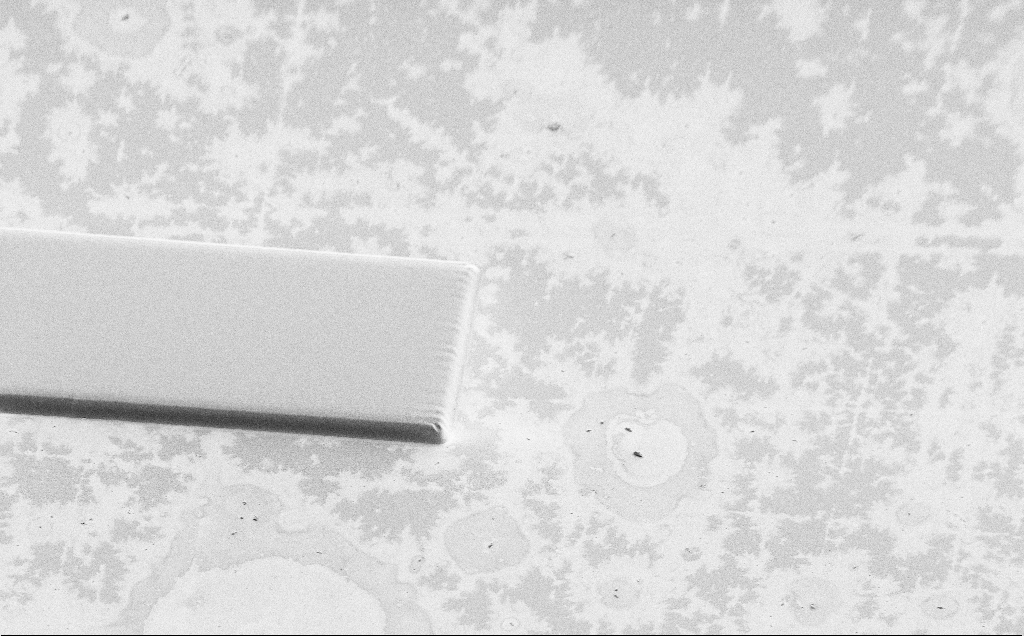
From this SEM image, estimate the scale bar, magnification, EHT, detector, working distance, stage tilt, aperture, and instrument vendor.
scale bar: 20000 nm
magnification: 1.01 K X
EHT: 1 kV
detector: SE2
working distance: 7 mm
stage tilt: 45°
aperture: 30 µm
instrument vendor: Zeiss